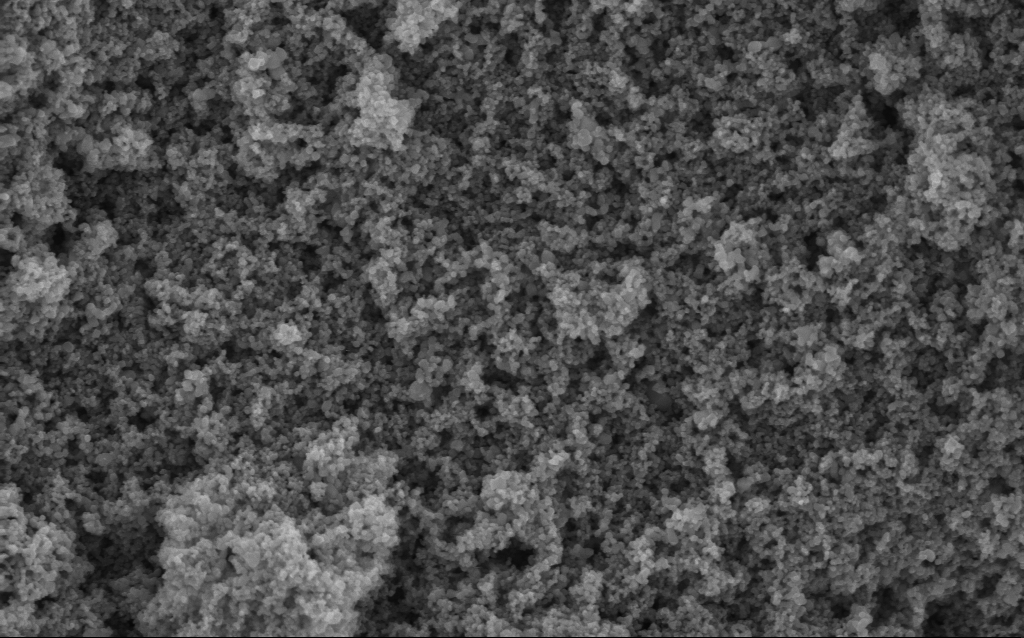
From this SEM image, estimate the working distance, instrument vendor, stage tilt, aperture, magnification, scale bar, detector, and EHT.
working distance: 4.6 mm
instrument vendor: Zeiss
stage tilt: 0°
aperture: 30 µm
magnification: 68.75 K X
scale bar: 1000 nm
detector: InLens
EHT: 5 kV